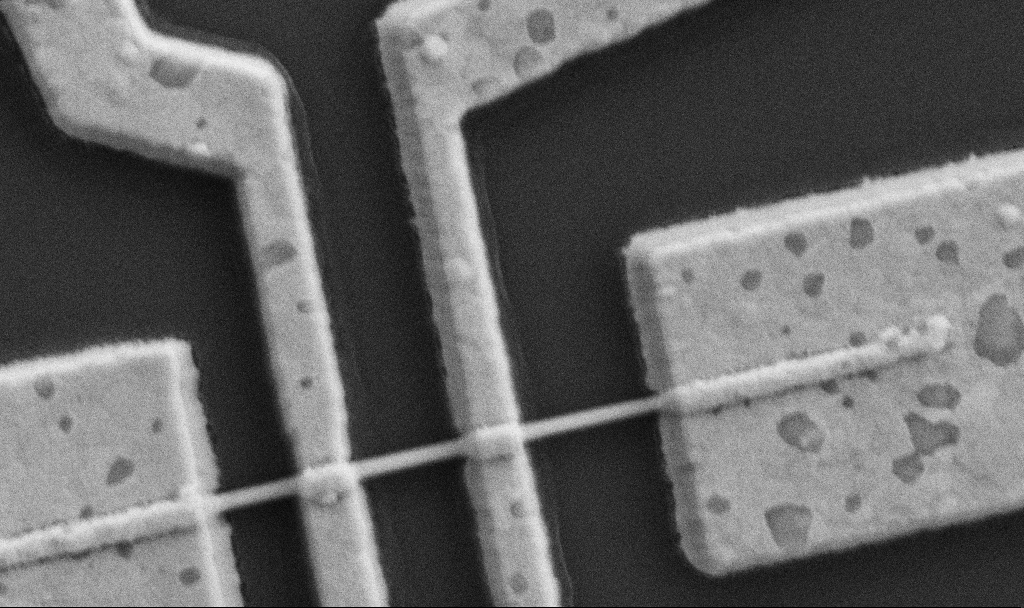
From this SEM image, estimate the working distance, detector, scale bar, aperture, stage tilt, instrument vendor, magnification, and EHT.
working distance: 9.7 mm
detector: SE2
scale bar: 1000 nm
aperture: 30 µm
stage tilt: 0°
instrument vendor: Zeiss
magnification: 60 K X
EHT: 5 kV